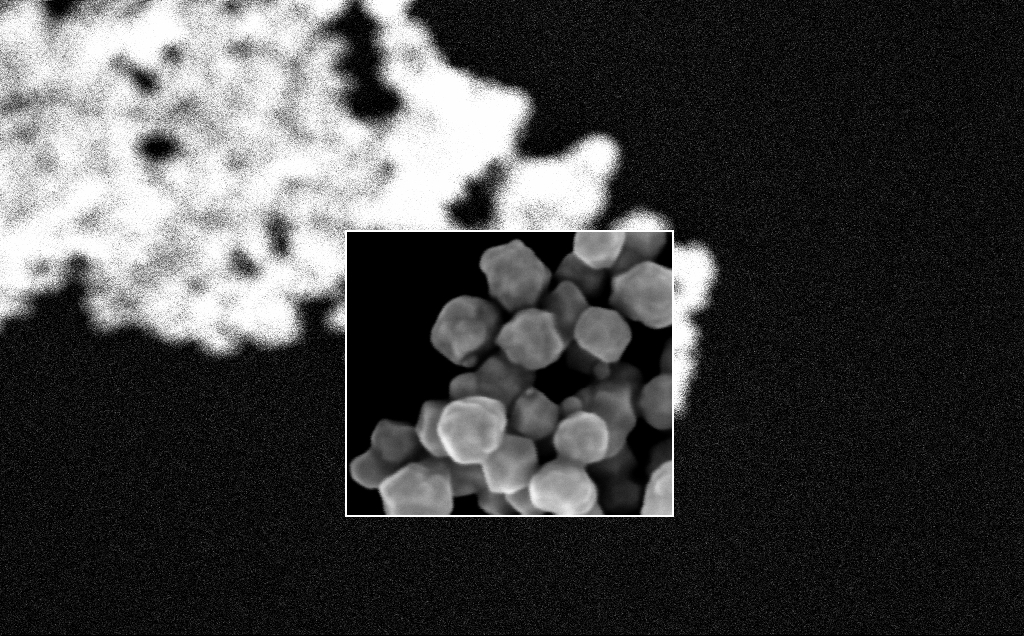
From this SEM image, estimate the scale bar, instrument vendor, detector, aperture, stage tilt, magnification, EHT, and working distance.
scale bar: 200 nm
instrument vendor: Zeiss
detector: InLens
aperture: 30 µm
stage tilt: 0°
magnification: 295.03 K X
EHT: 10 kV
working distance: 3 mm